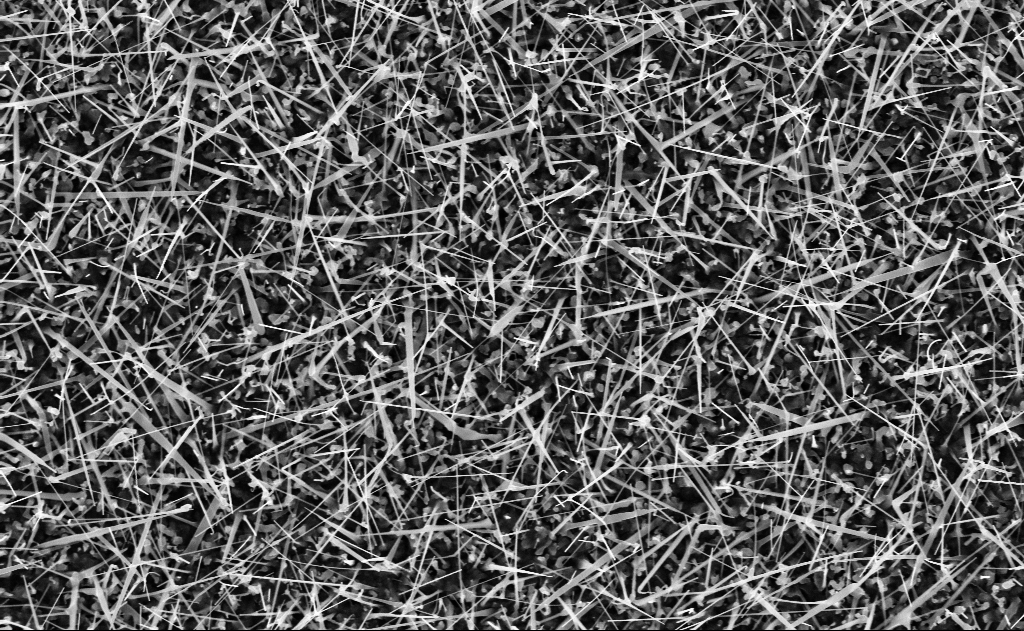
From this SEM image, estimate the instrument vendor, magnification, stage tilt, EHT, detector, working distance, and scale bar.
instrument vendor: Zeiss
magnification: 20 K X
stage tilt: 0°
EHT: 10 kV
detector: InLens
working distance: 12 mm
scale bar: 2000 nm